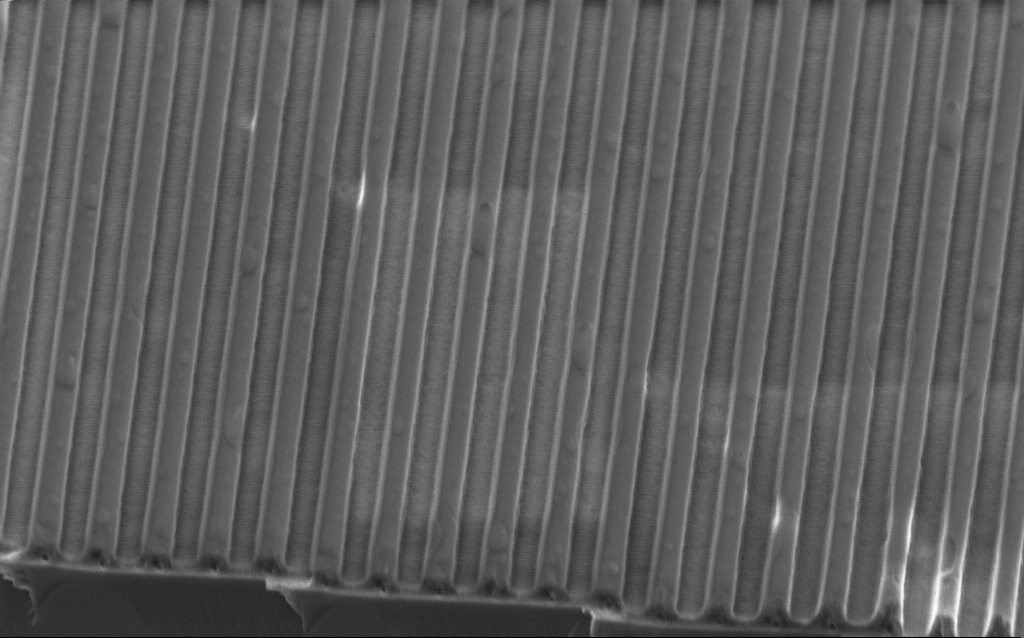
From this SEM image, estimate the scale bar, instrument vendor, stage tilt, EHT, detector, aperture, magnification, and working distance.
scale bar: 1000 nm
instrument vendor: Zeiss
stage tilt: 45°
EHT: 2 kV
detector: InLens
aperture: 30 µm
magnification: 41.53 K X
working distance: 3.8 mm